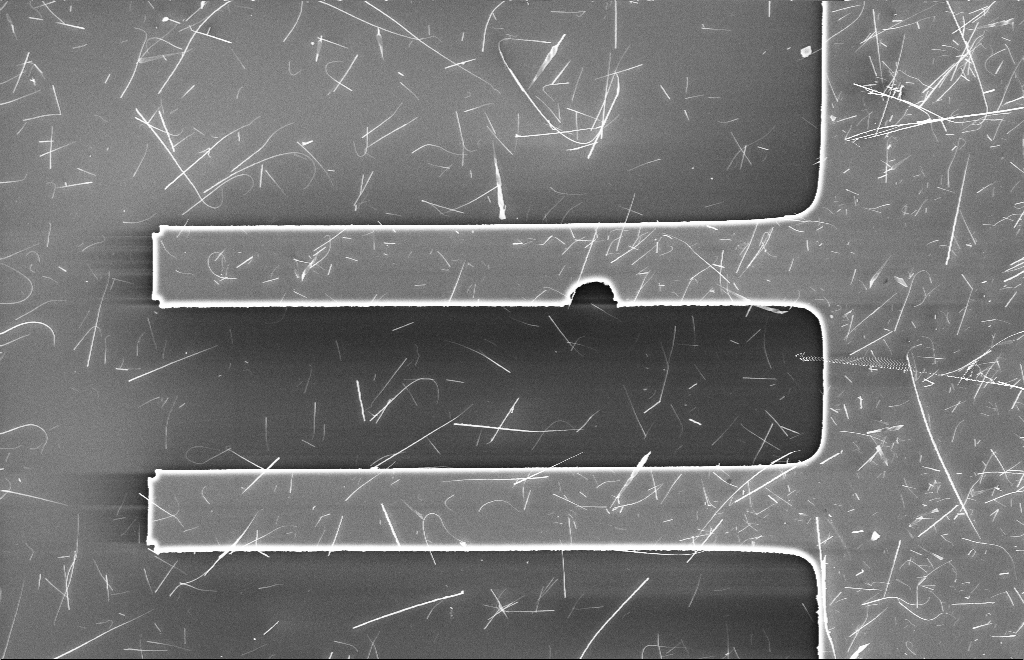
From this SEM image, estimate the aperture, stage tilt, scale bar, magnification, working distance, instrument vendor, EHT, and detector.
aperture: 20 µm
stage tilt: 0°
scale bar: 10000 nm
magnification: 2.5 K X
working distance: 6 mm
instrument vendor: Zeiss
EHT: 10 kV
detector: InLens